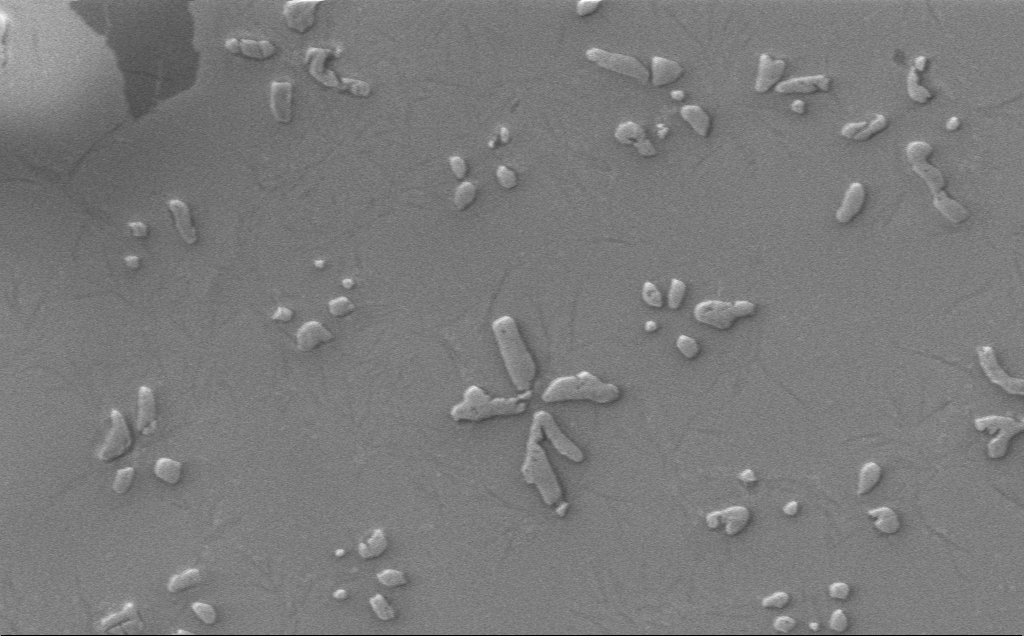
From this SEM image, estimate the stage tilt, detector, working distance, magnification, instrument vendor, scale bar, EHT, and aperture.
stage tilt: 0°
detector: InLens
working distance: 4 mm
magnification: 5.8 K X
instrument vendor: Zeiss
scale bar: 10000 nm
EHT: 1 kV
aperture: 30 µm